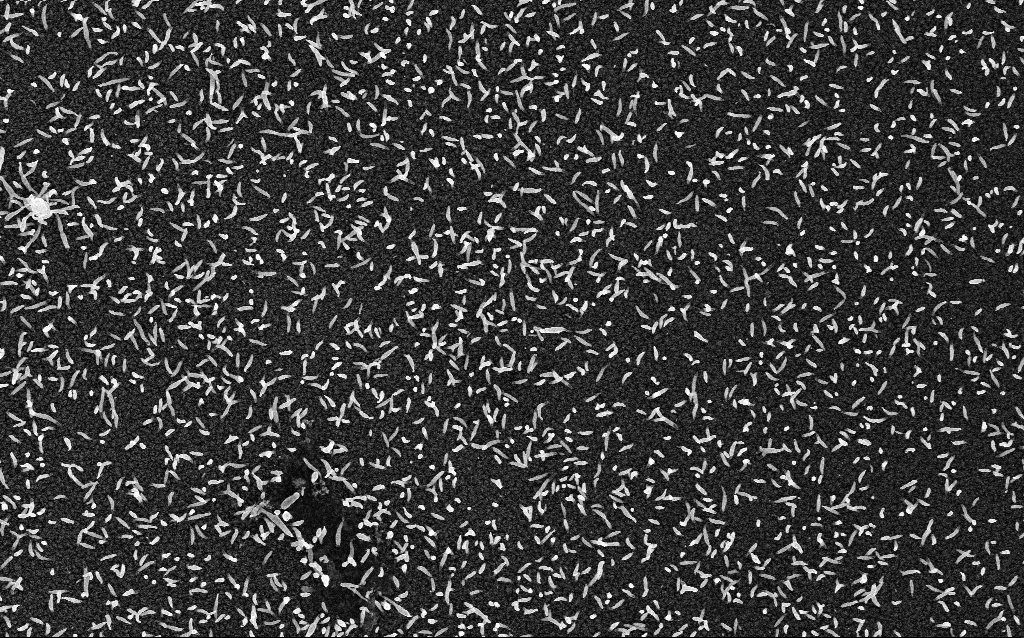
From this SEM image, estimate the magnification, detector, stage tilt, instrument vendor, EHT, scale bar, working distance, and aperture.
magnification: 20 K X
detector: InLens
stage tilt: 0°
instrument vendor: Zeiss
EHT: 5 kV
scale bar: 1000 nm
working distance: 2.1 mm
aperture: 30 µm